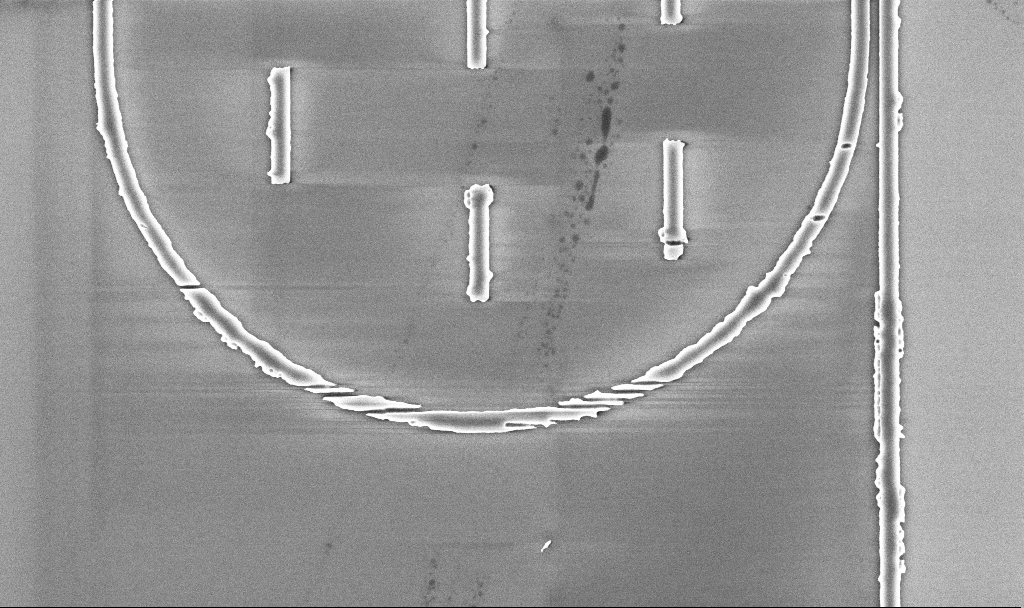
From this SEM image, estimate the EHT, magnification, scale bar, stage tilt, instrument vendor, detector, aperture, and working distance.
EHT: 5 kV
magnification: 14.41 K X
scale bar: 2000 nm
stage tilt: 0°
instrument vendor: Zeiss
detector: InLens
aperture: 30 µm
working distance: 5.2 mm